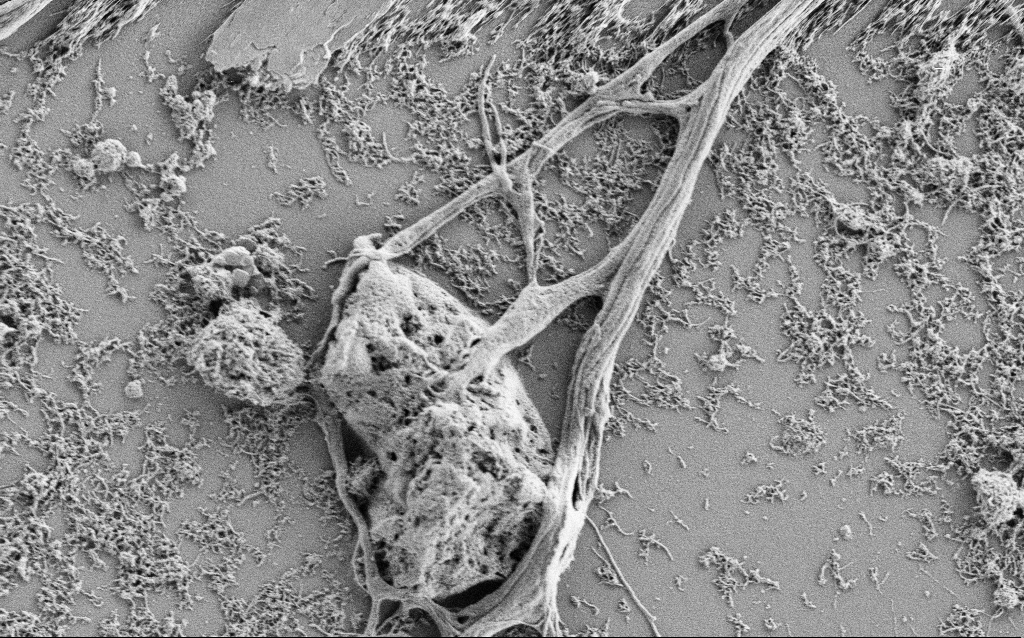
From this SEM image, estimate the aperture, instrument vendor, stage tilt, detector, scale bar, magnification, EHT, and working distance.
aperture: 30 µm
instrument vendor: Zeiss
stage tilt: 0°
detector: SE2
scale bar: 2000 nm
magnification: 10 K X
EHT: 1 kV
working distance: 4 mm